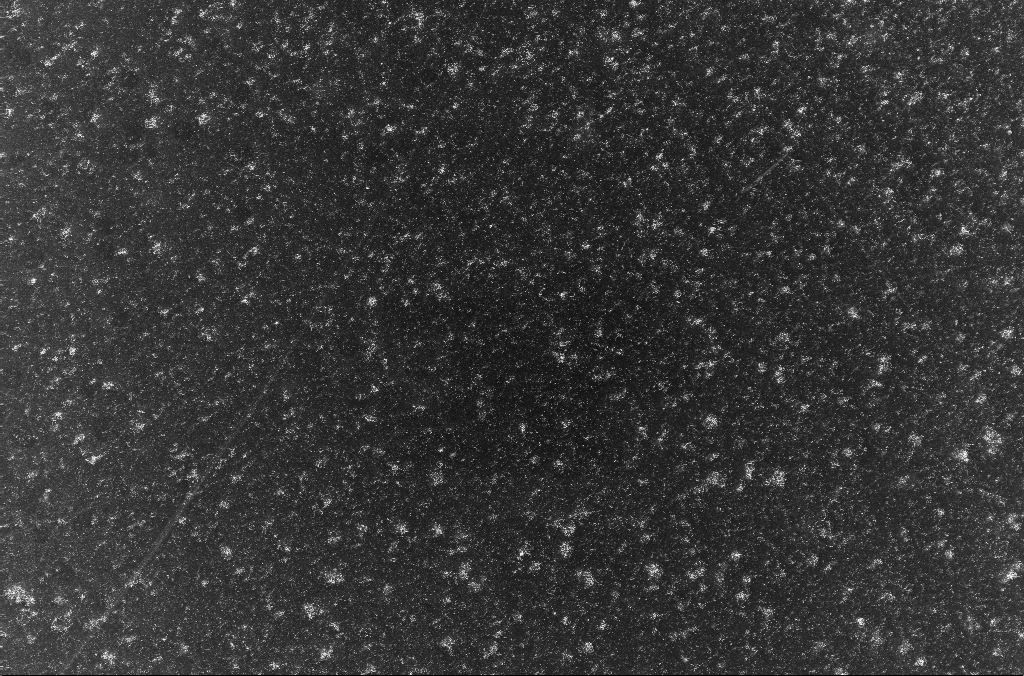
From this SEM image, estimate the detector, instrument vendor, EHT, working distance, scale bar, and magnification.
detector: InLens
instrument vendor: Zeiss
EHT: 10 kV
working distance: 3.3 mm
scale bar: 20000 nm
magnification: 1 K X